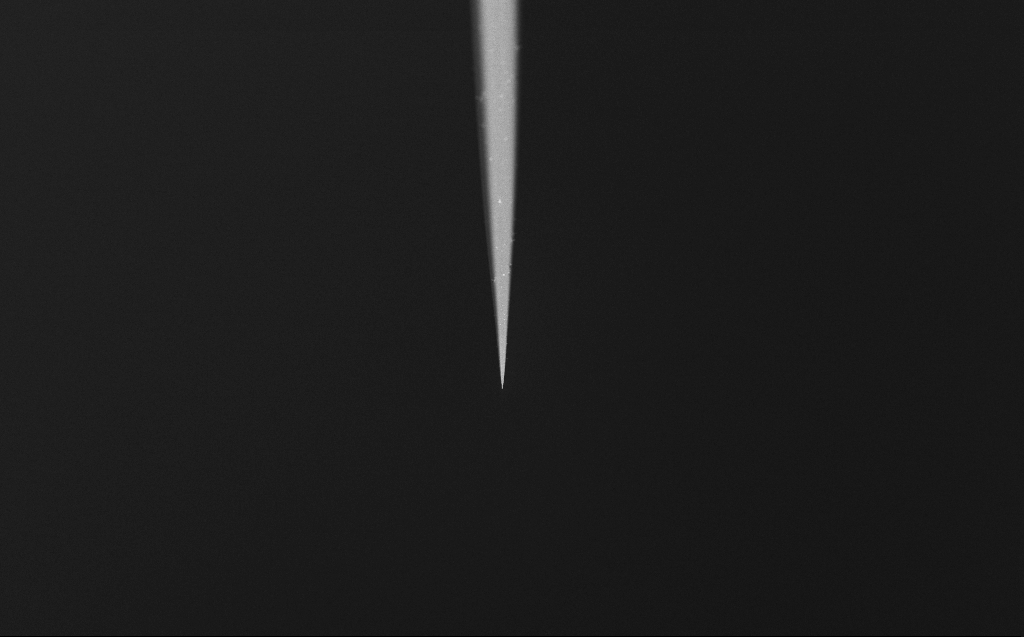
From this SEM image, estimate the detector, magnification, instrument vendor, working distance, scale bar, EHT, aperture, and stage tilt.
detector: InLens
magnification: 1 K X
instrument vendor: Zeiss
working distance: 3 mm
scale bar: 20000 nm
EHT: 5 kV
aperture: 30 µm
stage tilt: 45°